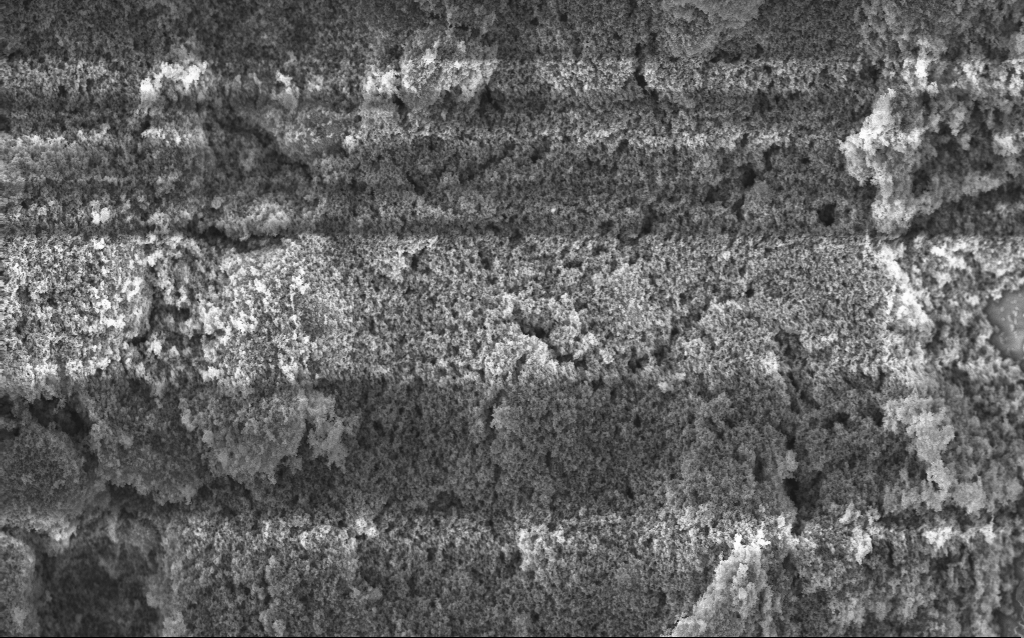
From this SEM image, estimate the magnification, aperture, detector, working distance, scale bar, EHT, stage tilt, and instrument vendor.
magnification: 15.33 K X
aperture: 30 µm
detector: InLens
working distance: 2.5 mm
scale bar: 1000 nm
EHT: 5 kV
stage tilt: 0°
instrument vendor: Zeiss